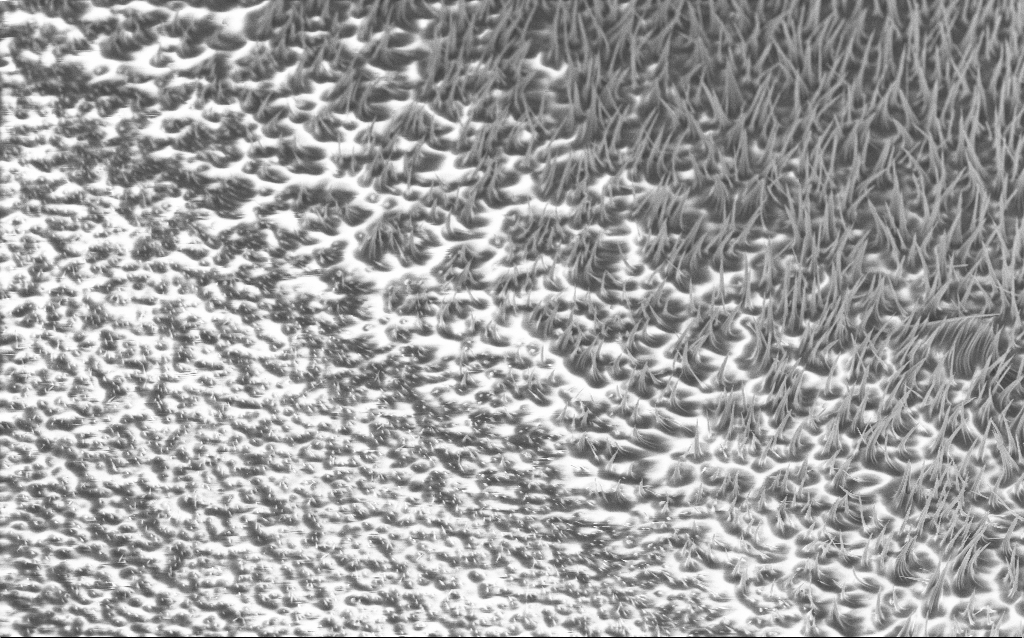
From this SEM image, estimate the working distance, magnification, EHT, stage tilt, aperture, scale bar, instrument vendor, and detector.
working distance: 6.3 mm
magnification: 5.29 K X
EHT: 5 kV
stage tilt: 40°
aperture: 30 µm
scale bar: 10000 nm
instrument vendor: Zeiss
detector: InLens